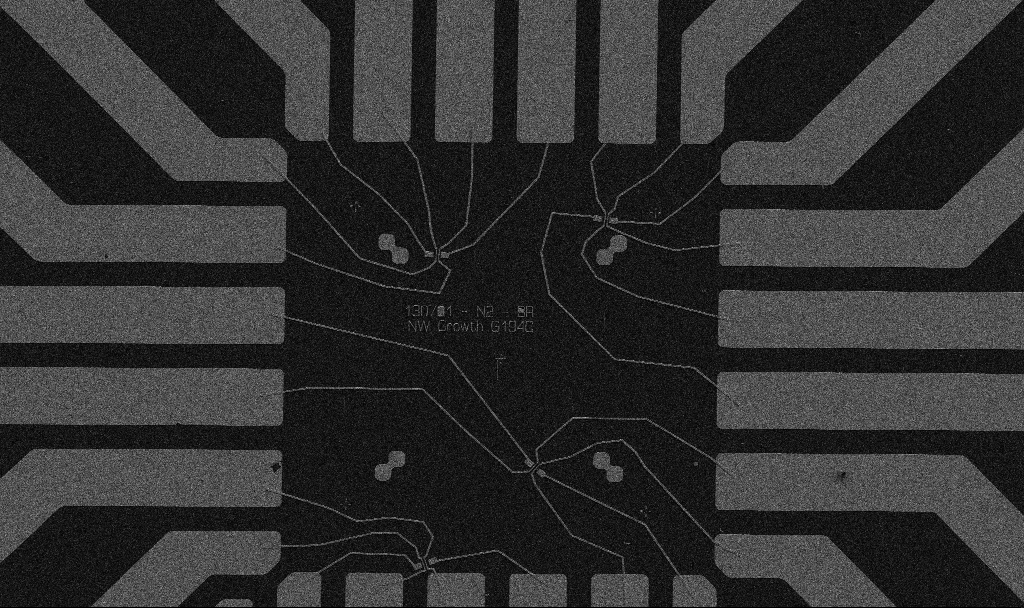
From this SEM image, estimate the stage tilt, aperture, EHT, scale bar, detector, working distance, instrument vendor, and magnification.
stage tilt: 0°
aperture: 30 µm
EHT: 5 kV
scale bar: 20000 nm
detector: SE2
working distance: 10.7 mm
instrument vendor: Zeiss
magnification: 1 K X